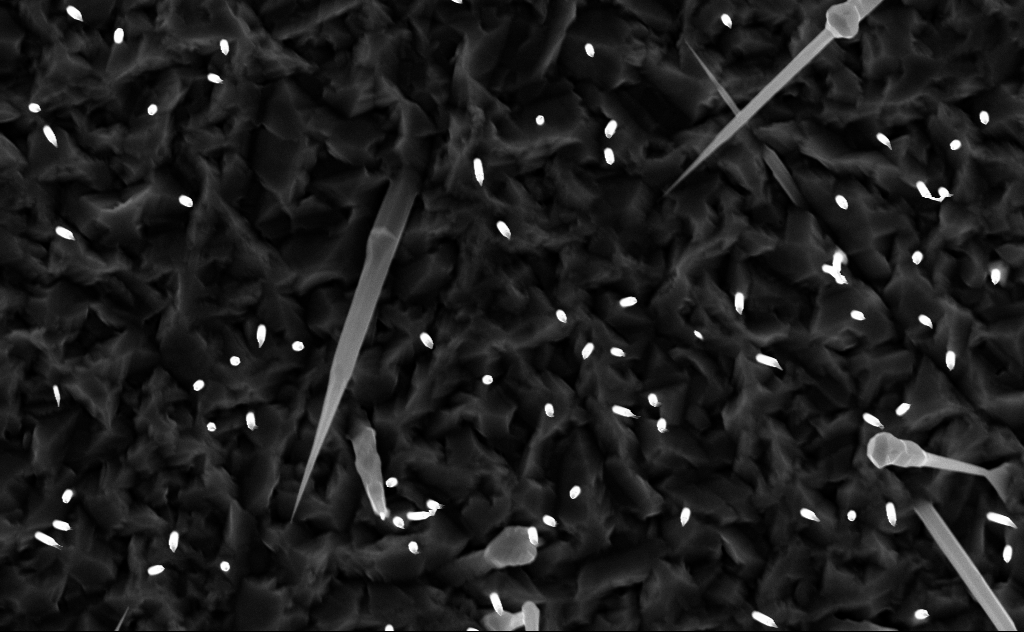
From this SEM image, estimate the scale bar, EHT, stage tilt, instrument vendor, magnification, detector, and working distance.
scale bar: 2000 nm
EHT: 10 kV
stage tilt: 0°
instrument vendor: Zeiss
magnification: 20 K X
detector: InLens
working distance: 6 mm